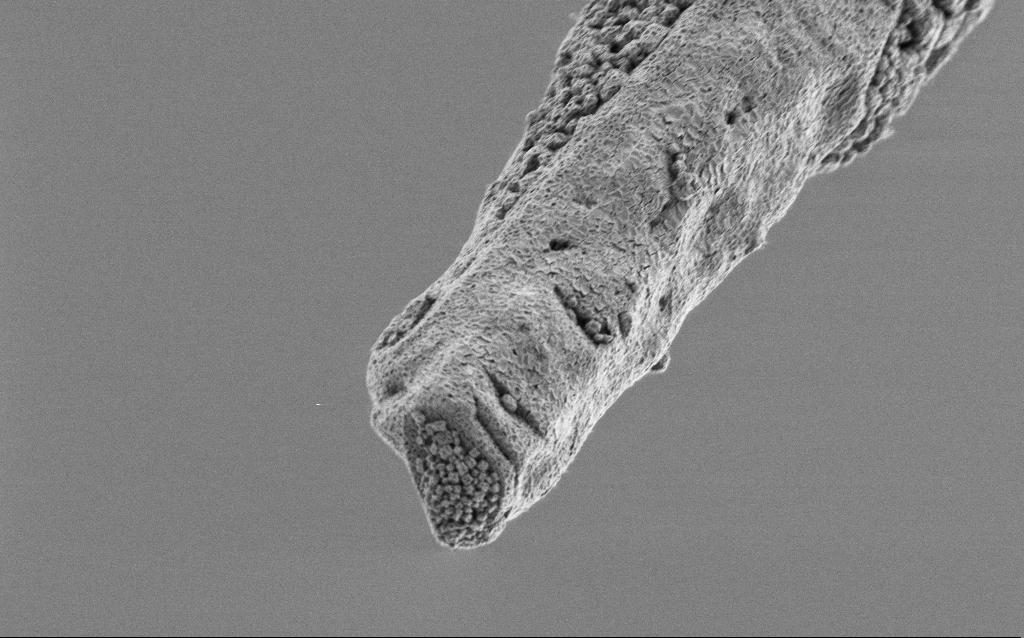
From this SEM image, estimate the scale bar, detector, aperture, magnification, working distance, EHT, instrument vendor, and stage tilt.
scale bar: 2000 nm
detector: SE2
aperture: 30 µm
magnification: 10 K X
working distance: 6.8 mm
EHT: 1 kV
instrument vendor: Zeiss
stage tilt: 45°